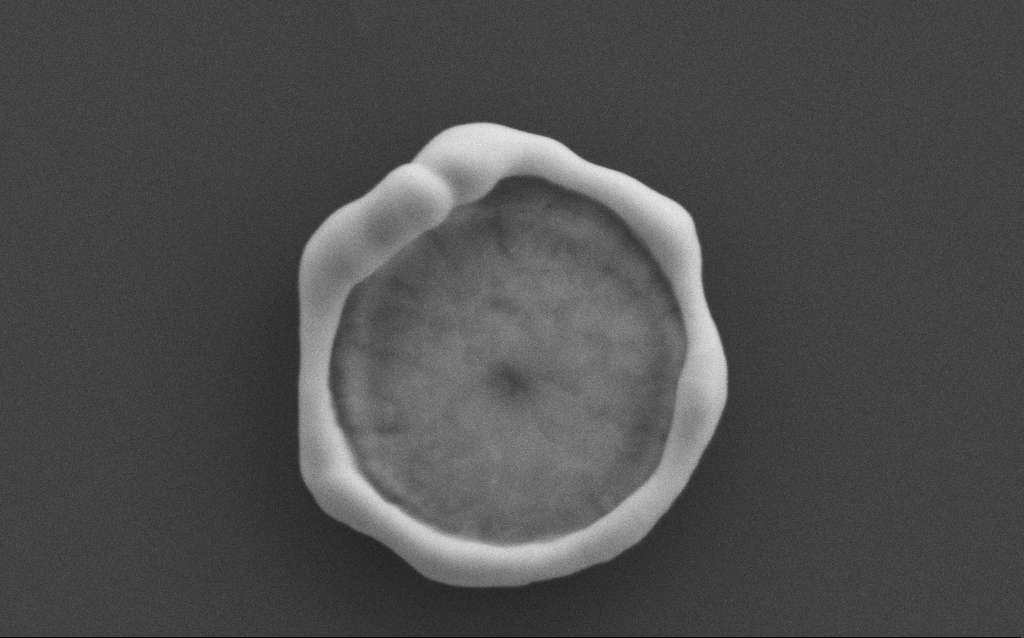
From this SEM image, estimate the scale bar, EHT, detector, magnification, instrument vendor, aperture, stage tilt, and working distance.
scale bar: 1000 nm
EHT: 10 kV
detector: SE2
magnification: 58.55 K X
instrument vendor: Zeiss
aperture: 30 µm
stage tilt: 0°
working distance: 4 mm